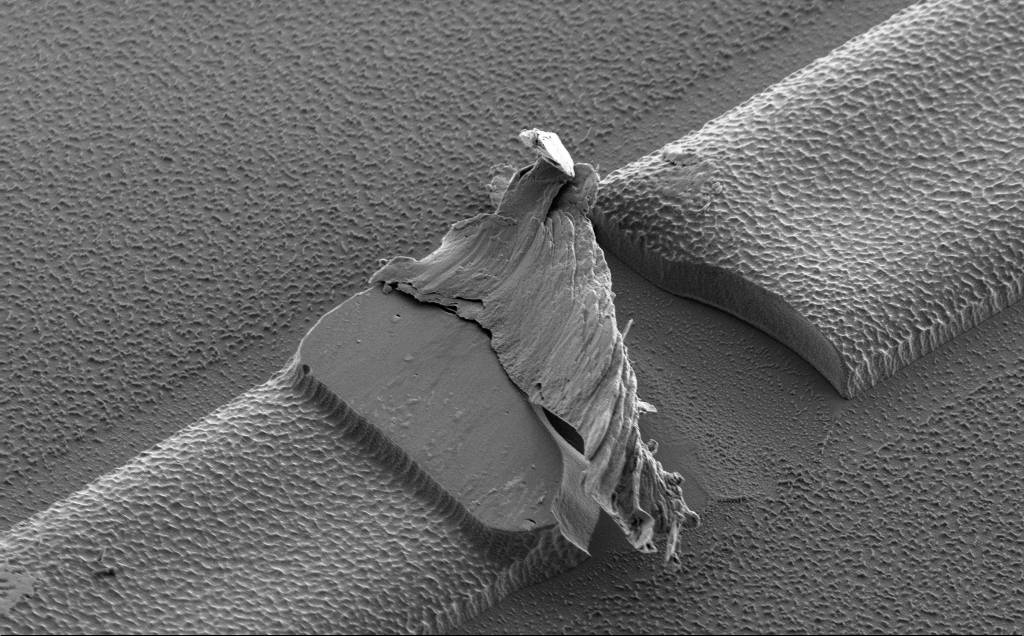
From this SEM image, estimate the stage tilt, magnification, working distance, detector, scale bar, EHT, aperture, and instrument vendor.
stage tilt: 43°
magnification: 8.79 K X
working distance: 10 mm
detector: SE2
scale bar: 2000 nm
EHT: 2 kV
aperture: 30 µm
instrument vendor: Zeiss